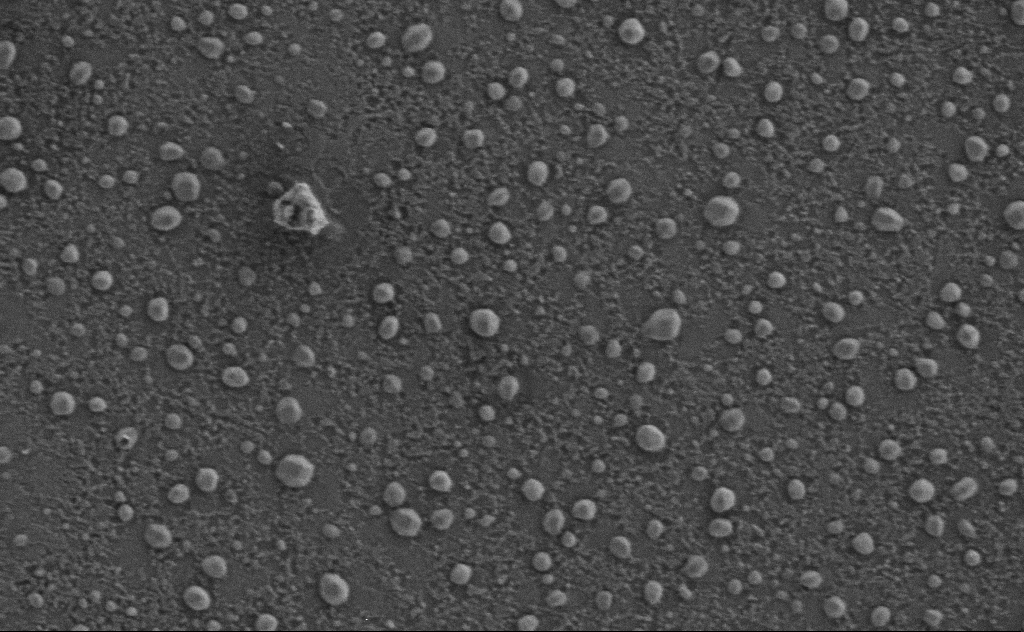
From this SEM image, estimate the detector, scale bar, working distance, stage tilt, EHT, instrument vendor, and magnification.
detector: SE2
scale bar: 200 nm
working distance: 4 mm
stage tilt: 0°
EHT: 1 kV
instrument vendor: Zeiss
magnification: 80 K X